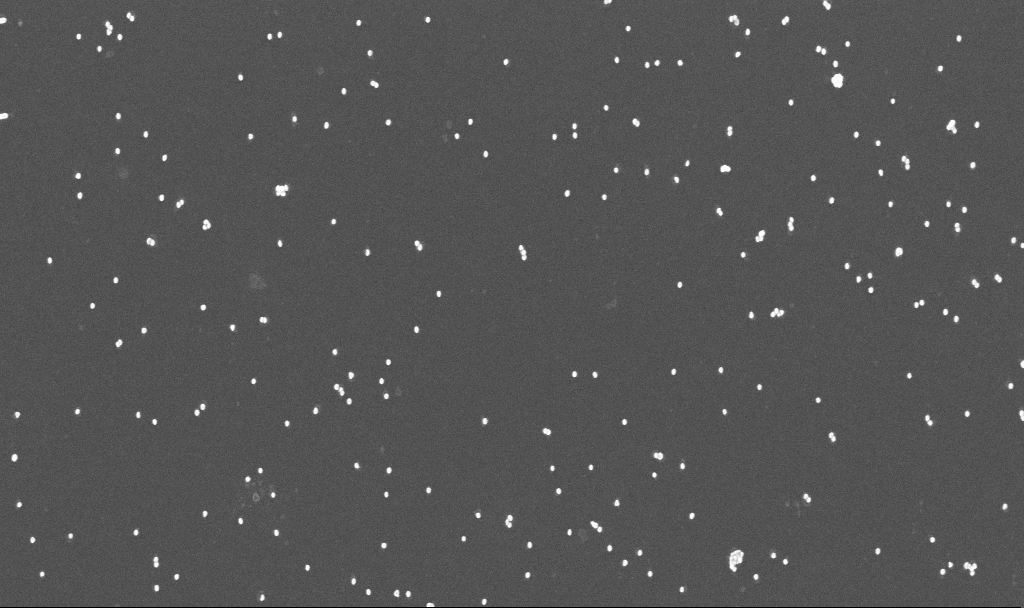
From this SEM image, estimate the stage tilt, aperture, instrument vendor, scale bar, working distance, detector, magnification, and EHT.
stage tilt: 0°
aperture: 30 µm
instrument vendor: Zeiss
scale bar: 1000 nm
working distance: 3.3 mm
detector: InLens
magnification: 70 K X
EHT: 10 kV